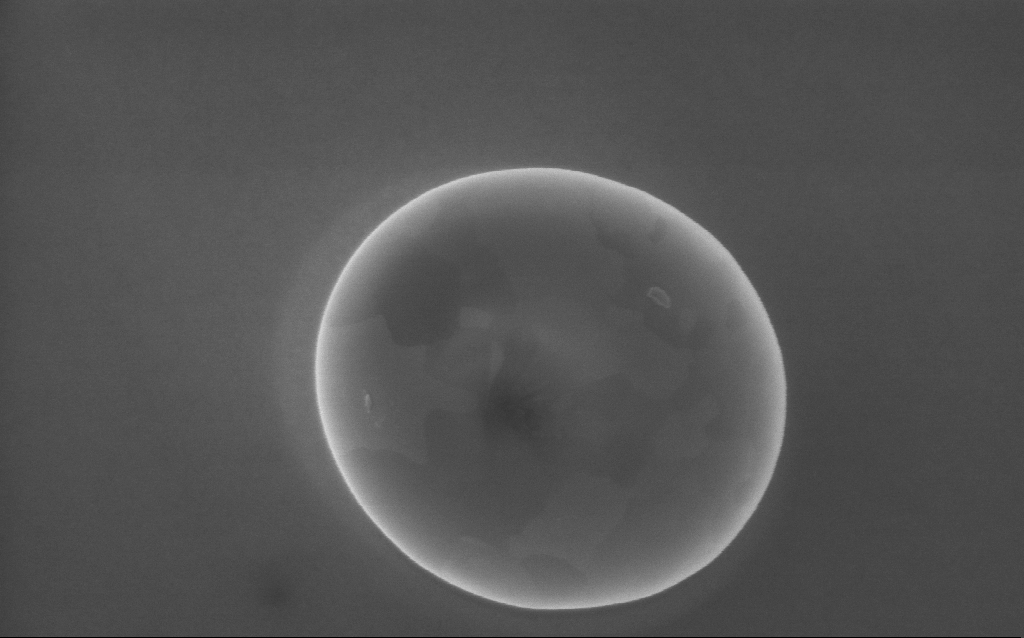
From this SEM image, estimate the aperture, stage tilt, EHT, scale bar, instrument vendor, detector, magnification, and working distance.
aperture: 30 µm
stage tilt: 0°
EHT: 5 kV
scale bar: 200 nm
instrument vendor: Zeiss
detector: InLens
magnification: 100 K X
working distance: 4 mm